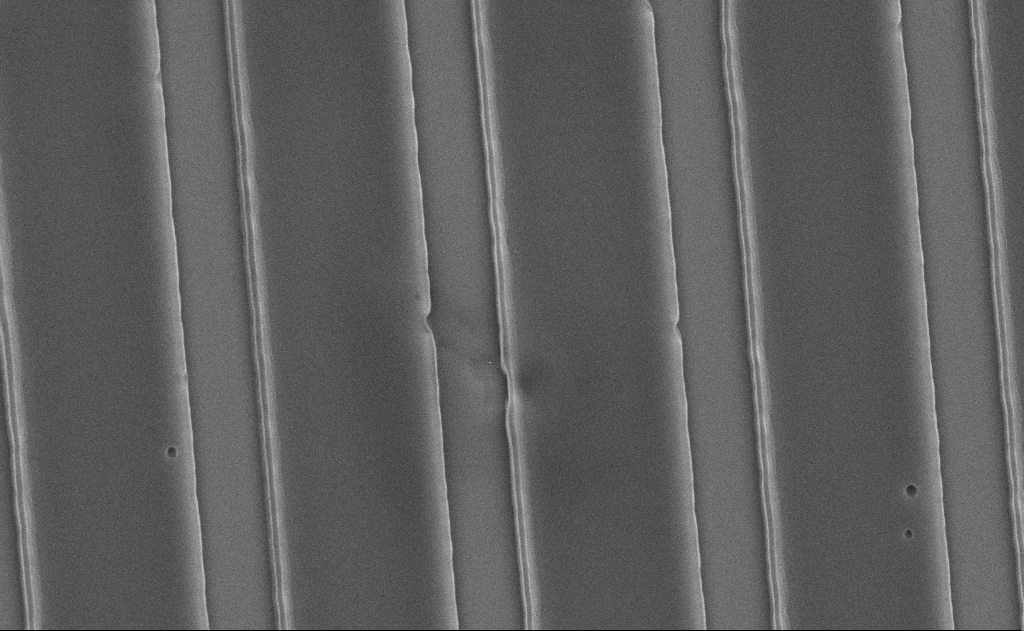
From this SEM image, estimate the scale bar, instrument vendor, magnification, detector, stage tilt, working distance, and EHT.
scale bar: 1000 nm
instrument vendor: Zeiss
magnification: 22.57 K X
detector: SE2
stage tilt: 0°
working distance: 12 mm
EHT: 5 kV